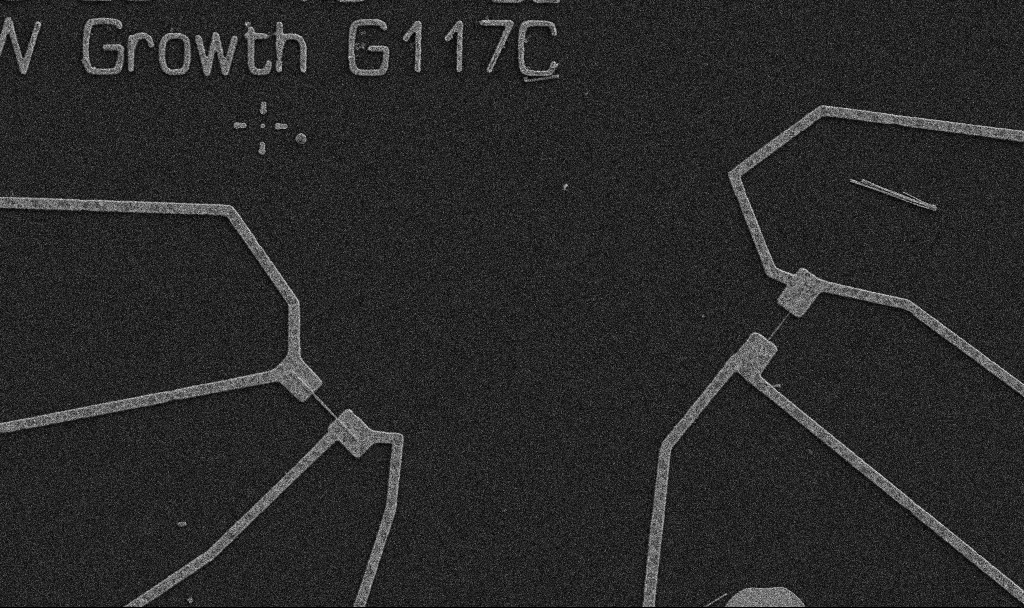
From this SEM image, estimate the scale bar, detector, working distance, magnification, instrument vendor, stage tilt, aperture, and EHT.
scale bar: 10000 nm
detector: SE2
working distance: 10.7 mm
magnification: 5 K X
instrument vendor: Zeiss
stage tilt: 0°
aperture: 30 µm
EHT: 5 kV